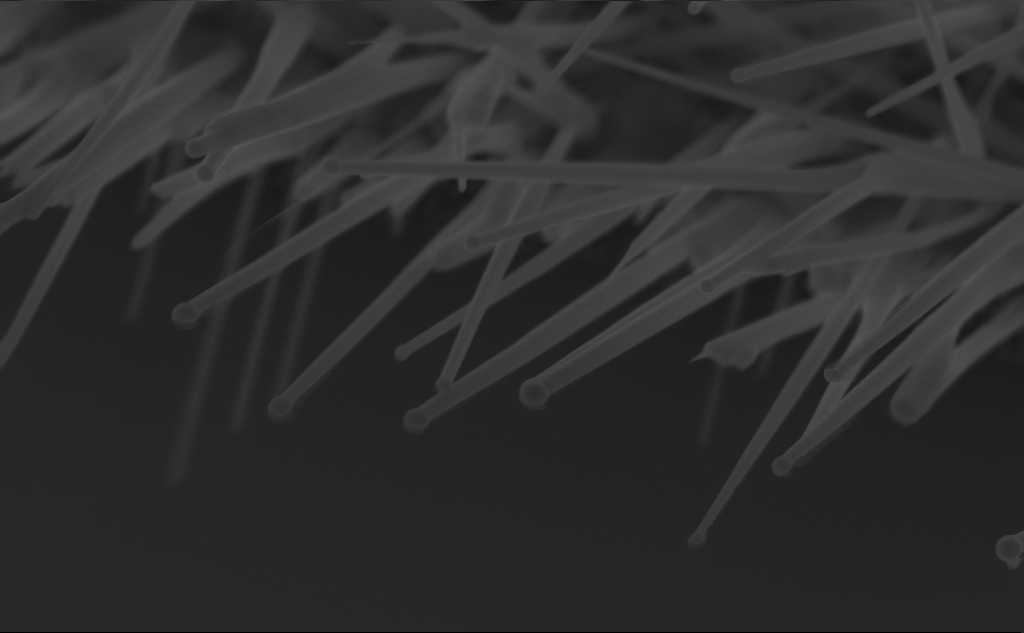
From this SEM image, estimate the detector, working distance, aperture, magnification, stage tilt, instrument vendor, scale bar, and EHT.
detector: InLens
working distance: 5 mm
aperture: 30 µm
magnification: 80.1 K X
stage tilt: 45°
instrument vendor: Zeiss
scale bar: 200 nm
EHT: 10 kV